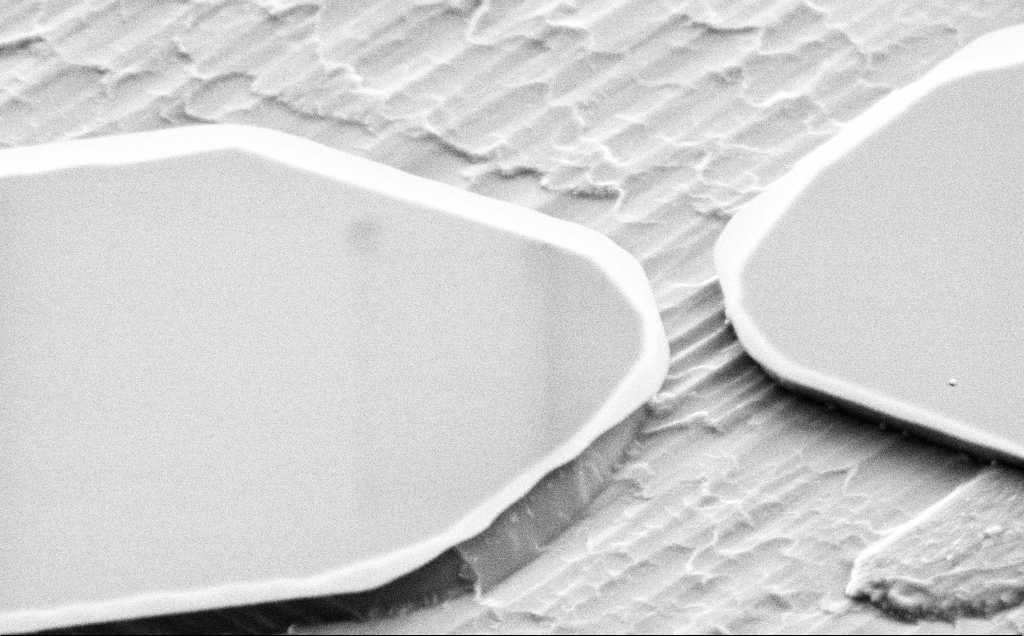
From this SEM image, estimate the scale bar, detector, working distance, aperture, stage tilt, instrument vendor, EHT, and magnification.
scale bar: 2000 nm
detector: SE2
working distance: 10 mm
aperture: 30 µm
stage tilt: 49.4°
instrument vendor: Zeiss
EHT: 5 kV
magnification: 13.79 K X